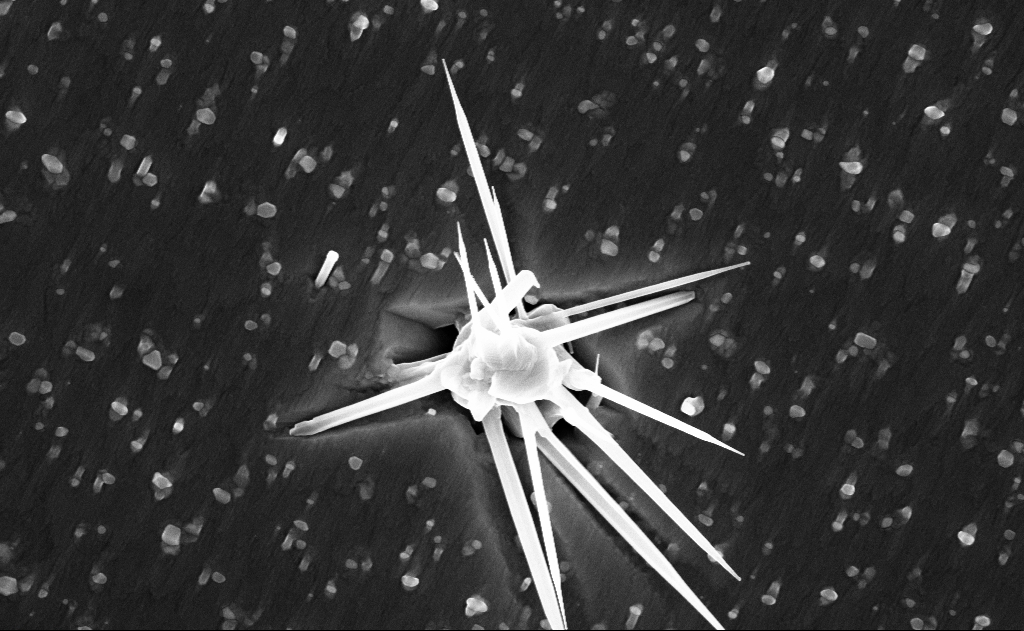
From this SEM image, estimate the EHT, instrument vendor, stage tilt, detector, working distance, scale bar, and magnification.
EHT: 10 kV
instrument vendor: Zeiss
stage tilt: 0°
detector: InLens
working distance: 9 mm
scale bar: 1000 nm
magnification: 40 K X